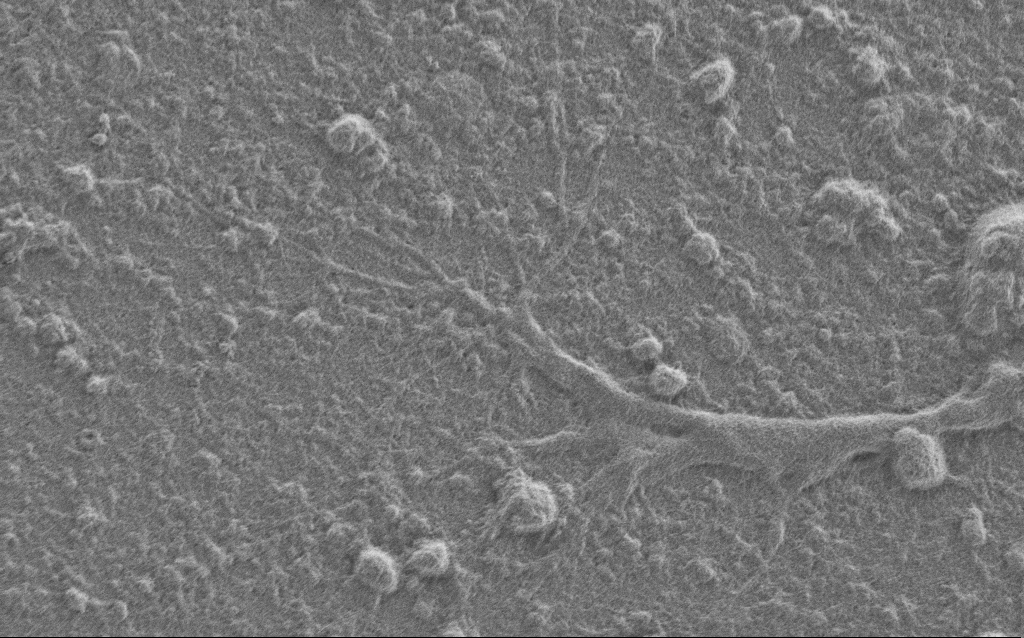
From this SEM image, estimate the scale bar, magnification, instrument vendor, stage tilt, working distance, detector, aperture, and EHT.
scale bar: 2000 nm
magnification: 7.5 K X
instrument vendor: Zeiss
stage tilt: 0°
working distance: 6 mm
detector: SE2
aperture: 30 µm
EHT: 1 kV